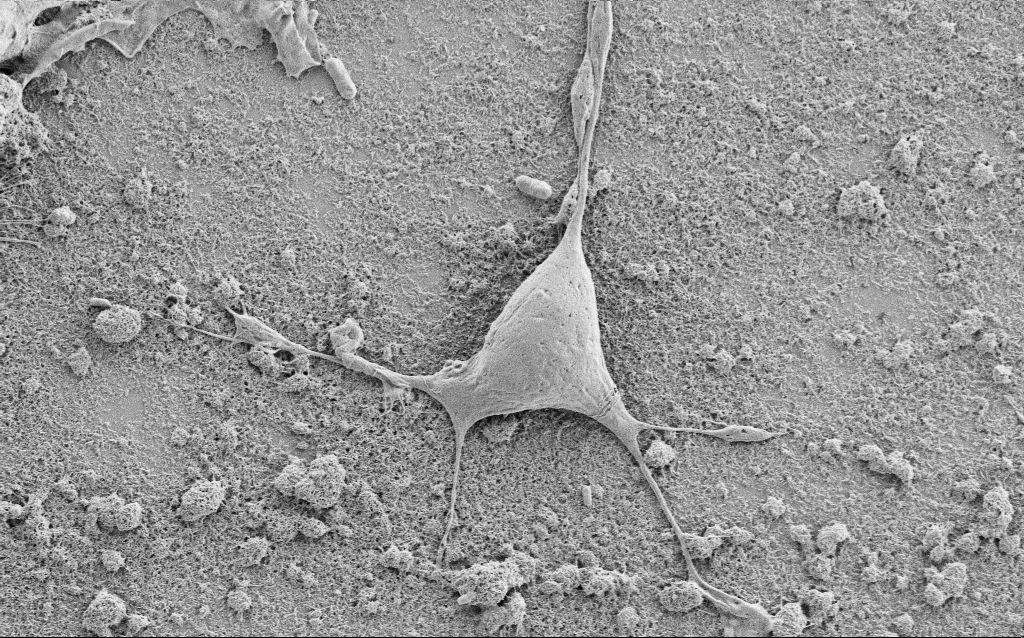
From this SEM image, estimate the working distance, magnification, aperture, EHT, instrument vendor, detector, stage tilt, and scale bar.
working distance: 6.7 mm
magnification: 5 K X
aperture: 30 µm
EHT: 1.5 kV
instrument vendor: Zeiss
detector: SE2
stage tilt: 0°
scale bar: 10000 nm